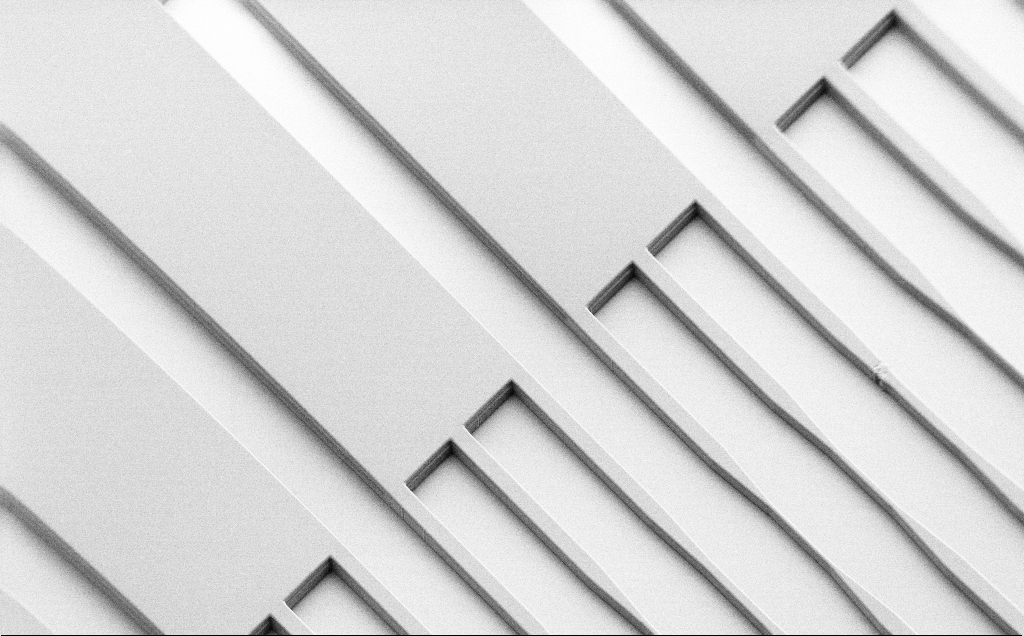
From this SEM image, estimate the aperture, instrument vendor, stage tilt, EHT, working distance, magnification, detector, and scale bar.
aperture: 30 µm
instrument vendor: Zeiss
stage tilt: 45°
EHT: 1.3 kV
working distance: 8 mm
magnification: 0.29 K X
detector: SE2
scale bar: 100000 nm